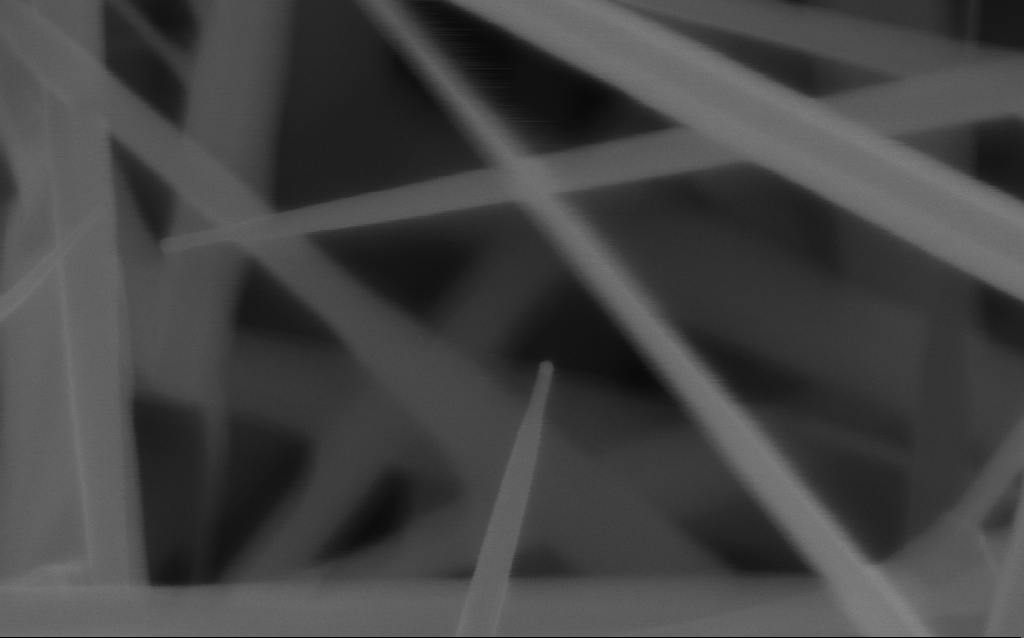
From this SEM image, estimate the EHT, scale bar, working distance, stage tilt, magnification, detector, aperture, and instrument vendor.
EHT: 10 kV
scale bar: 200 nm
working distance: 4 mm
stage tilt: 0°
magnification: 183.16 K X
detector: InLens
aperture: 30 µm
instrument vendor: Zeiss